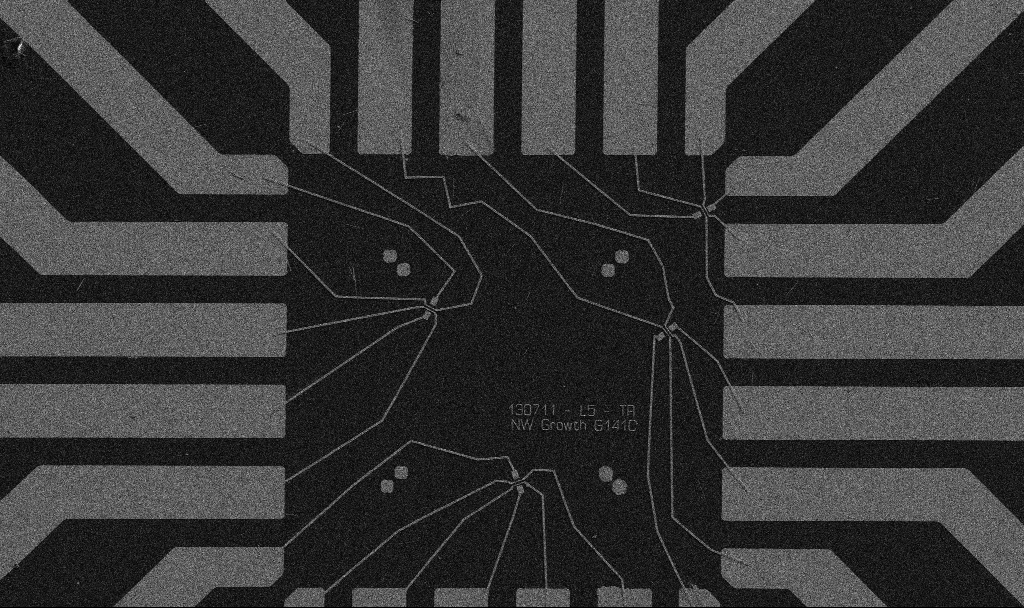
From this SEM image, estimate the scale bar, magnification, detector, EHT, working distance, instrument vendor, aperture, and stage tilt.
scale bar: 20000 nm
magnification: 1 K X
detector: SE2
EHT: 5 kV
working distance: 10.7 mm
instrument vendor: Zeiss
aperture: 30 µm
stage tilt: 0°